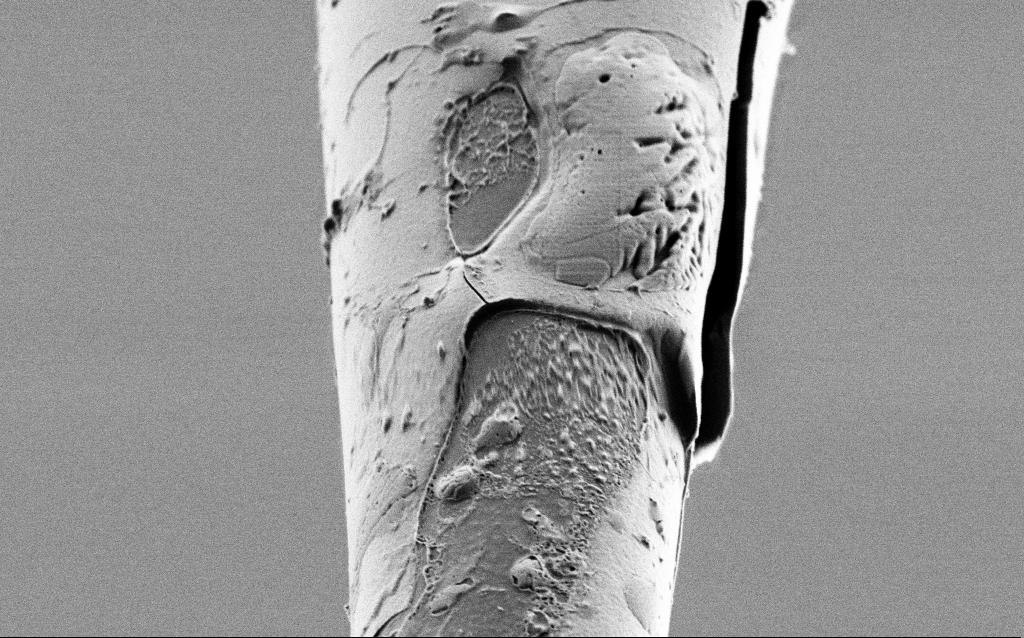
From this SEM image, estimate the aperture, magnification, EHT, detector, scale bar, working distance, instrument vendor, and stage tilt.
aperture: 30 µm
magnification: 25 K X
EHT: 1 kV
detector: SE2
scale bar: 1000 nm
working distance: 6.5 mm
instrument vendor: Zeiss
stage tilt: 45°